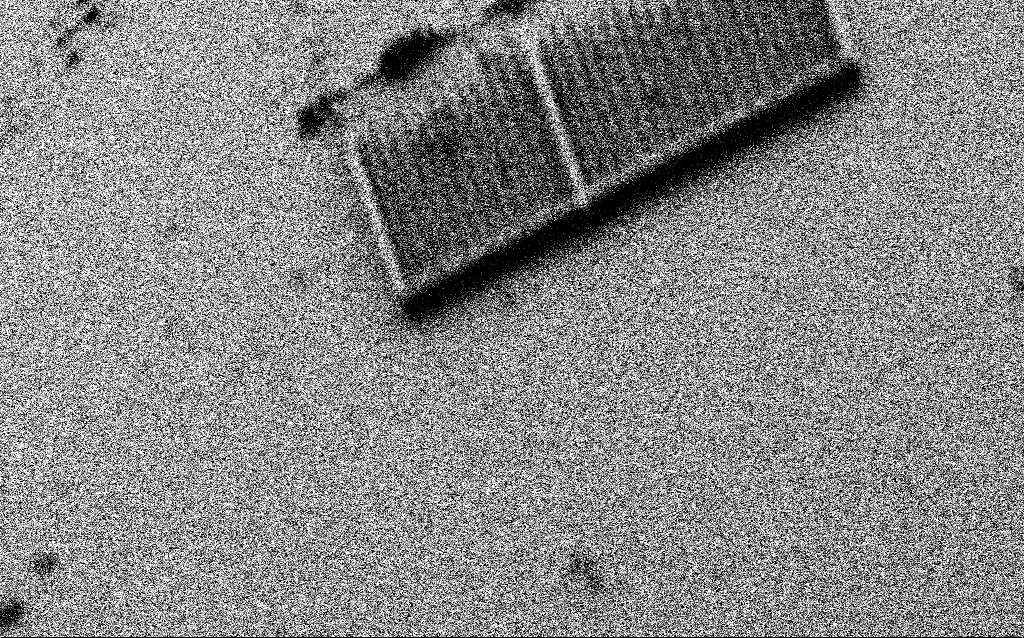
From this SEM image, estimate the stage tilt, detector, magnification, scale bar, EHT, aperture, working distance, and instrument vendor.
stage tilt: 50°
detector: SE2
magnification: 0.641 K X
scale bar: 100000 nm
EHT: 1.5 kV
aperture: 30 µm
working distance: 3.8 mm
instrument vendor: Zeiss